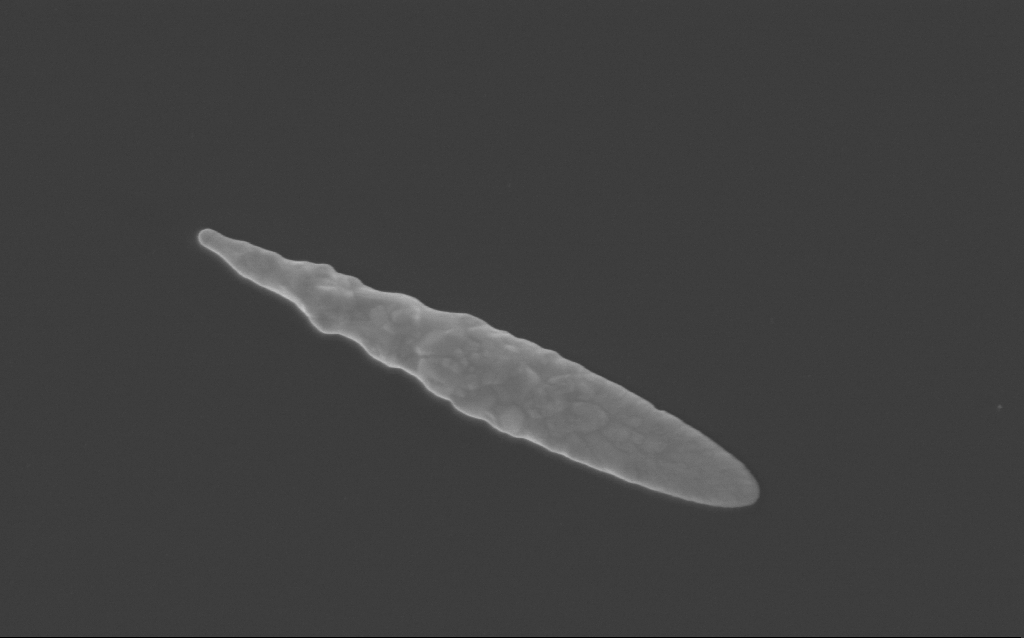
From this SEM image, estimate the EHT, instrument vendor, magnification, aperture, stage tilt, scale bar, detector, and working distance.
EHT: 3 kV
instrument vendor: Zeiss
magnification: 54.27 K X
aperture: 30 µm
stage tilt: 0°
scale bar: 1000 nm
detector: InLens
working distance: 3 mm